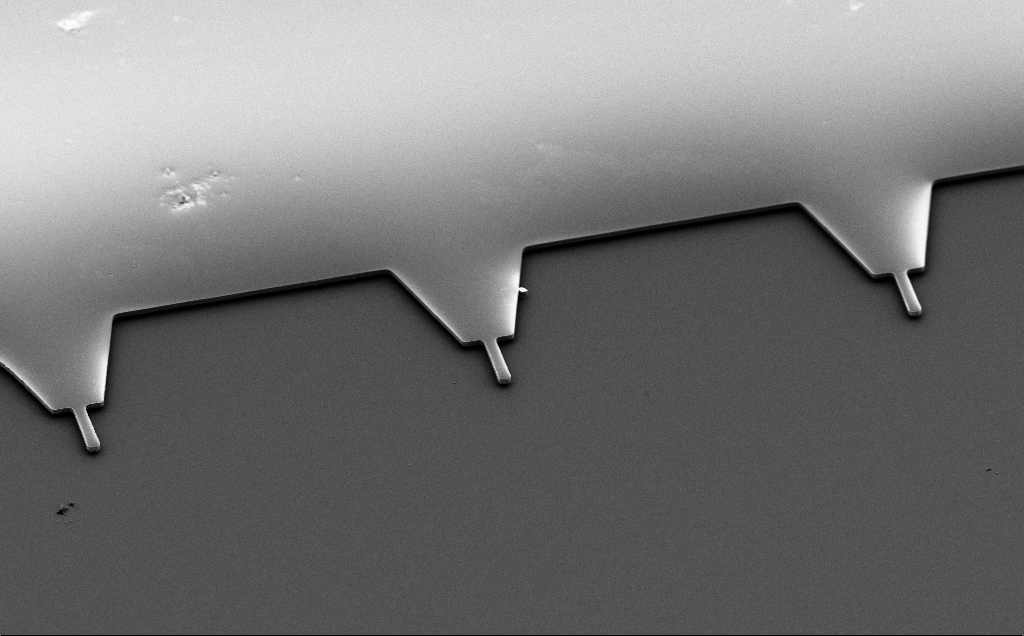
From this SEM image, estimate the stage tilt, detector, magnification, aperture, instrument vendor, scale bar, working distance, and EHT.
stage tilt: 50°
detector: SE2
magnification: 0.975 K X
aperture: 30 µm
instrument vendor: Zeiss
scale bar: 20000 nm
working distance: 10 mm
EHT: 5 kV